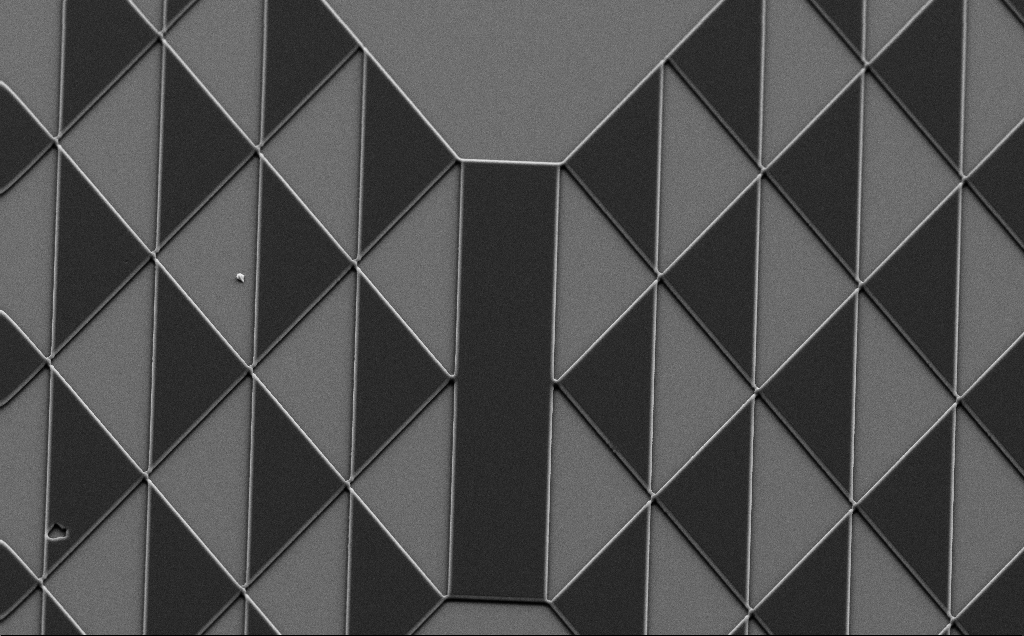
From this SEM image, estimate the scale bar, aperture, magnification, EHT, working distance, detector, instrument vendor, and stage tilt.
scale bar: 20000 nm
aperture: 30 µm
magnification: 1.05 K X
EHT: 10 kV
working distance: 8 mm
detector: SE2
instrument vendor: Zeiss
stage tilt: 35°